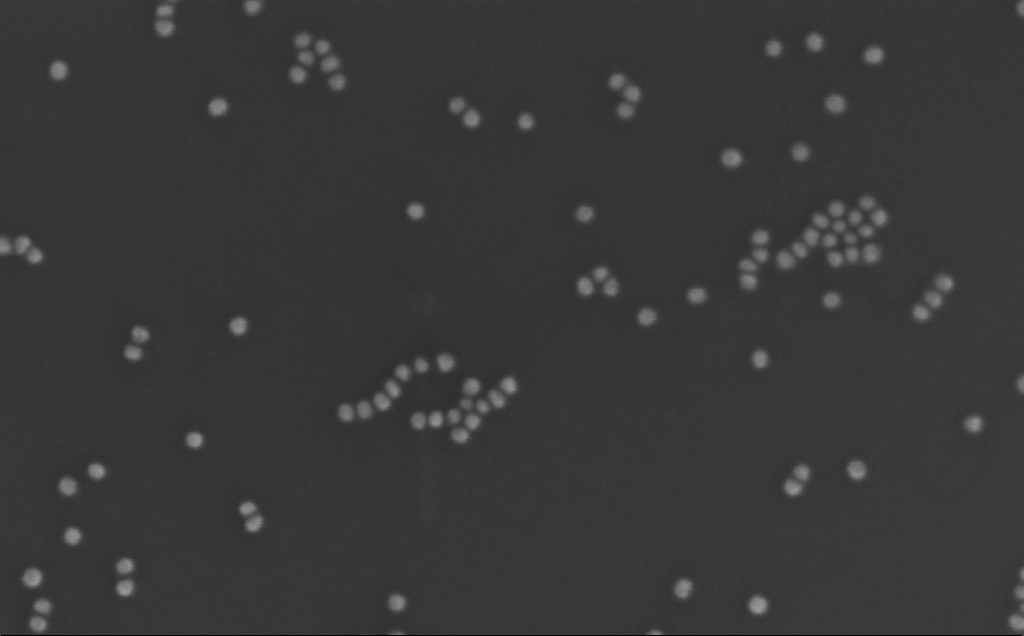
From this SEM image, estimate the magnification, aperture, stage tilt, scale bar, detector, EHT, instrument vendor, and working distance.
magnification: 366.22 K X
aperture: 30 µm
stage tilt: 0°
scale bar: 100 nm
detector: InLens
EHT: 10 kV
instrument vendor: Zeiss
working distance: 3 mm